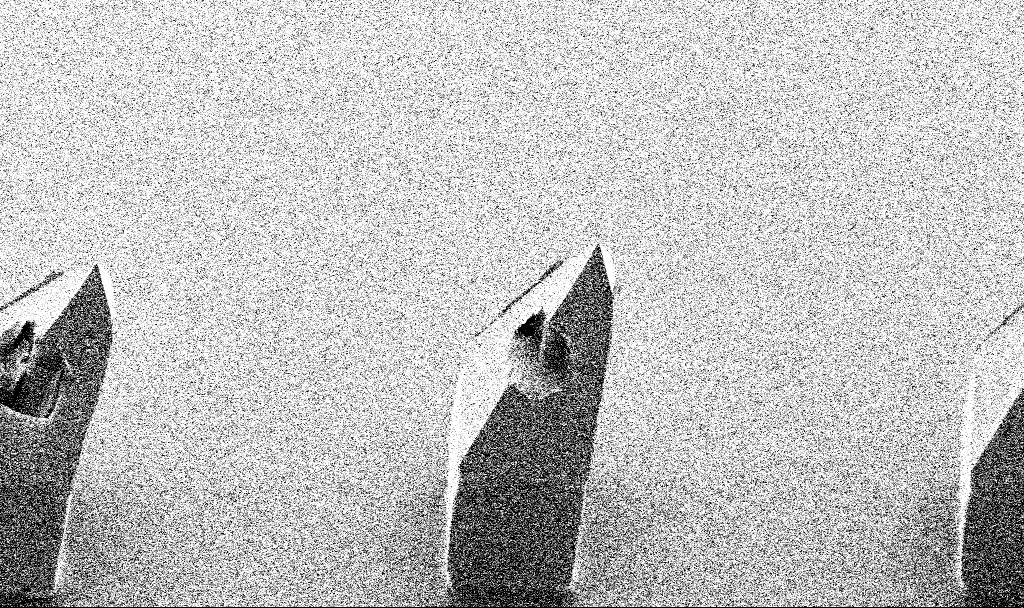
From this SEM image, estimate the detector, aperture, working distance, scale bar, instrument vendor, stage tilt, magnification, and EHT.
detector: SE2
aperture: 30 µm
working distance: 8.5 mm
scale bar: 100000 nm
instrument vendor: Zeiss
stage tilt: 45°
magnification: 0.24 K X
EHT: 3 kV